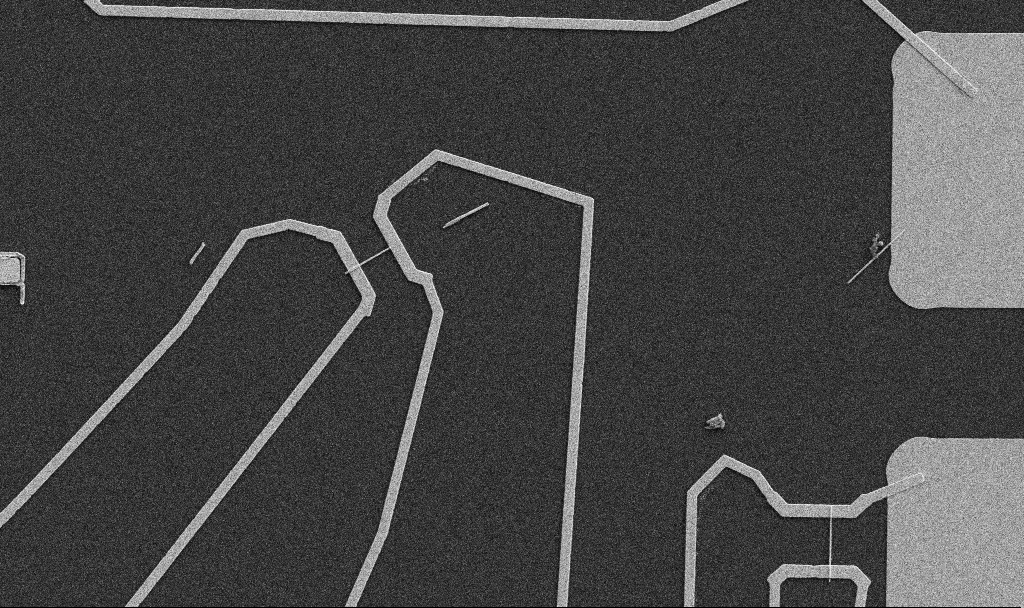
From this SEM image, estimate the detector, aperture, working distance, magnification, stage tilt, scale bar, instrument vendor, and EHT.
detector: SE2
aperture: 30 µm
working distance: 10.7 mm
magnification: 5 K X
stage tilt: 0°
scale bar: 10000 nm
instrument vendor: Zeiss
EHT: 5 kV